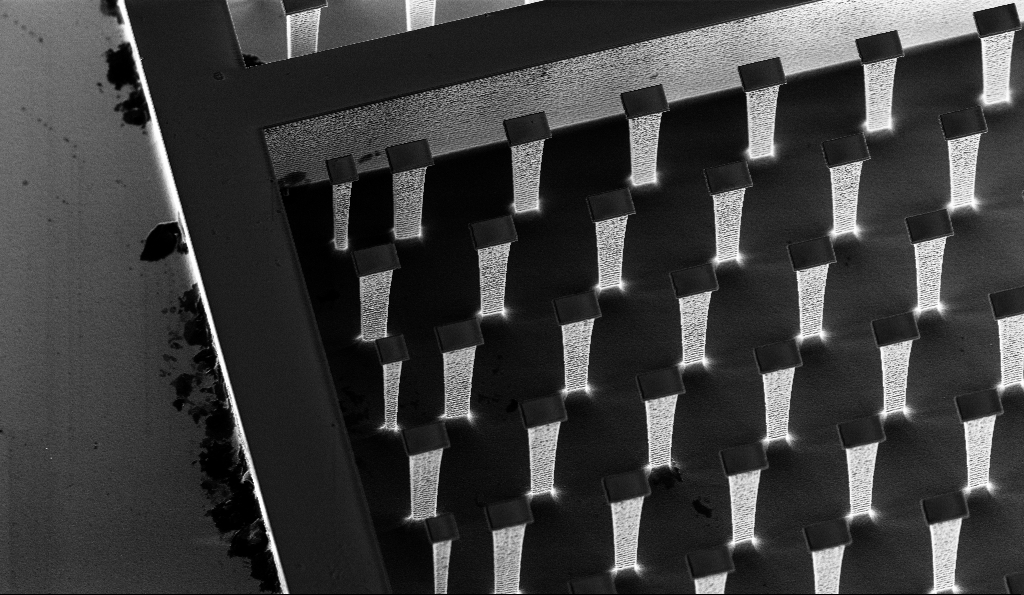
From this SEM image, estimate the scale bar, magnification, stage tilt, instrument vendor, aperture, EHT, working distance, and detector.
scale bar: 10000 nm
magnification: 3.68 K X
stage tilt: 30°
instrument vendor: Zeiss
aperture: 30 µm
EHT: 3 kV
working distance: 5.6 mm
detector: InLens